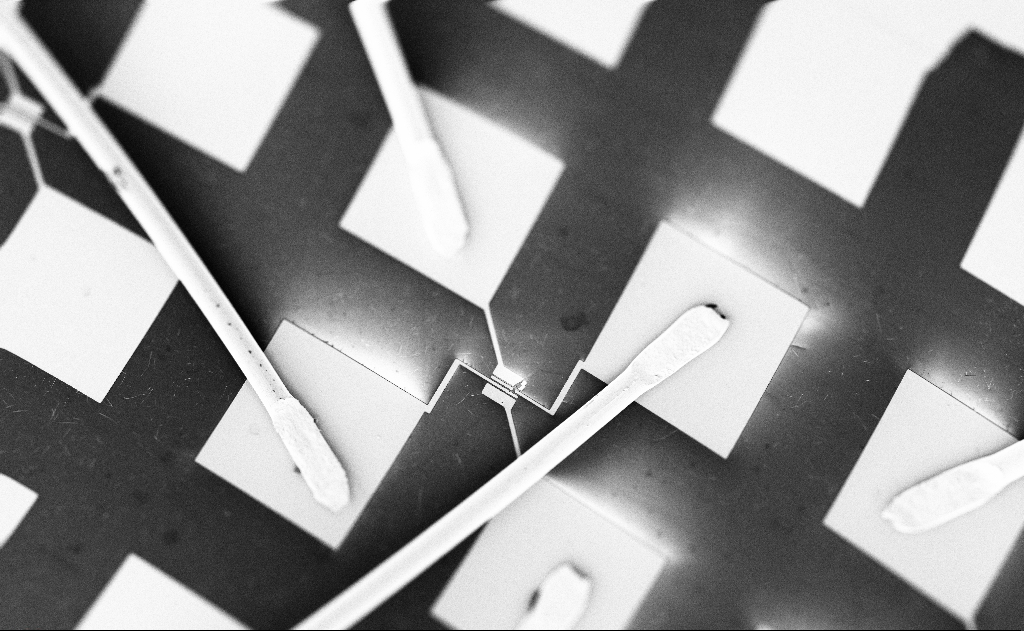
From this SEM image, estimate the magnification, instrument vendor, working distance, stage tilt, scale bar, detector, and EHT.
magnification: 0.408 K X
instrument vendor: Zeiss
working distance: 20 mm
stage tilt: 0°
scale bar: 100000 nm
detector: SE2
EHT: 5 kV